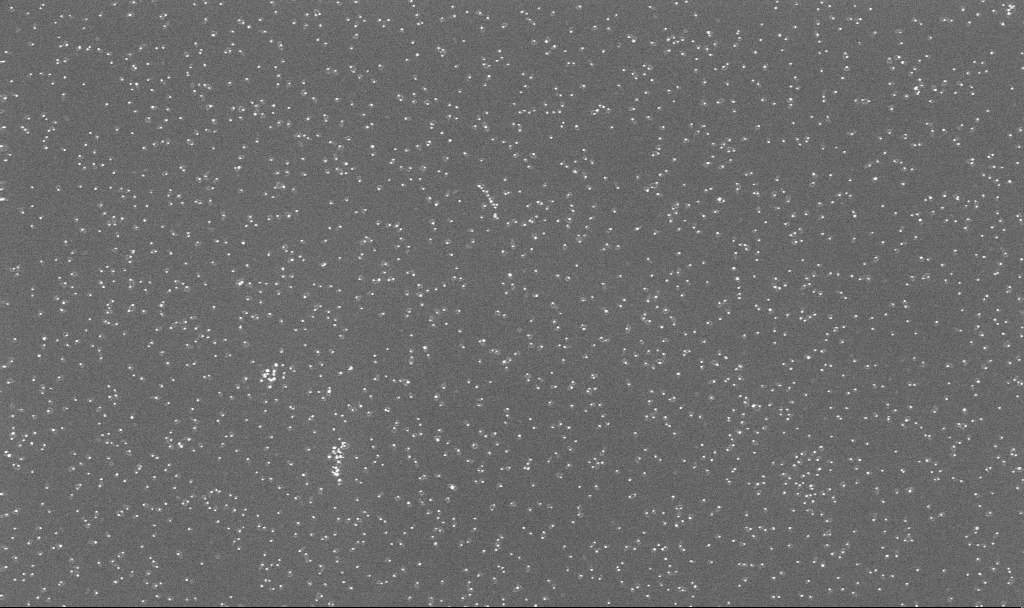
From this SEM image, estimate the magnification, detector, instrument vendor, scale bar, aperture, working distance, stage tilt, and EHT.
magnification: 70 K X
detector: InLens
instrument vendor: Zeiss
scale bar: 1000 nm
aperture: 30 µm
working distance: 3.4 mm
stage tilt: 0°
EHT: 10 kV